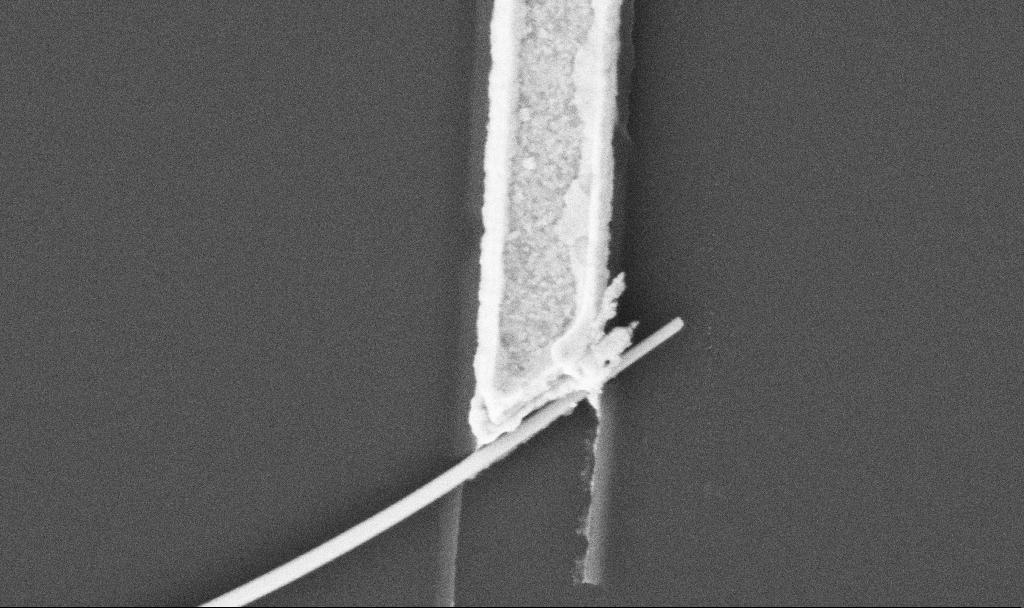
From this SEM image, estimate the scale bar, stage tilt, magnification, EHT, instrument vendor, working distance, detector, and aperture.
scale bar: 1000 nm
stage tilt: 0°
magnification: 60 K X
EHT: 5 kV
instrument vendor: Zeiss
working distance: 10.7 mm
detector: SE2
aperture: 30 µm